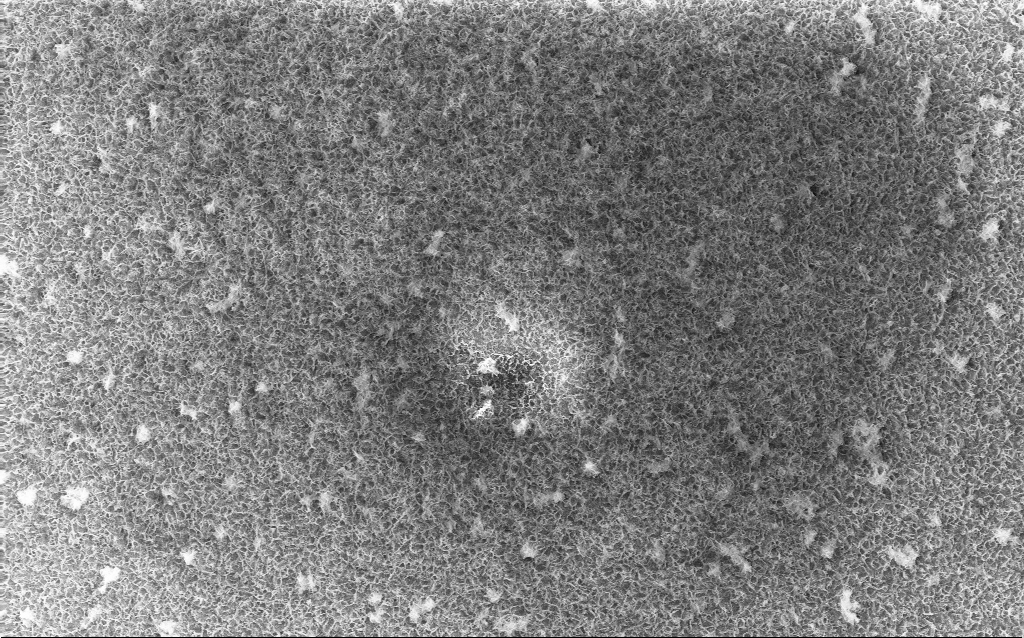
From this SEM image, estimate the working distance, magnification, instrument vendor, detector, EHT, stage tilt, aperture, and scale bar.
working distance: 2.8 mm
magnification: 2 K X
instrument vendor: Zeiss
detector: InLens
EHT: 10 kV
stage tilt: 0°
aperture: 30 µm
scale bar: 20000 nm